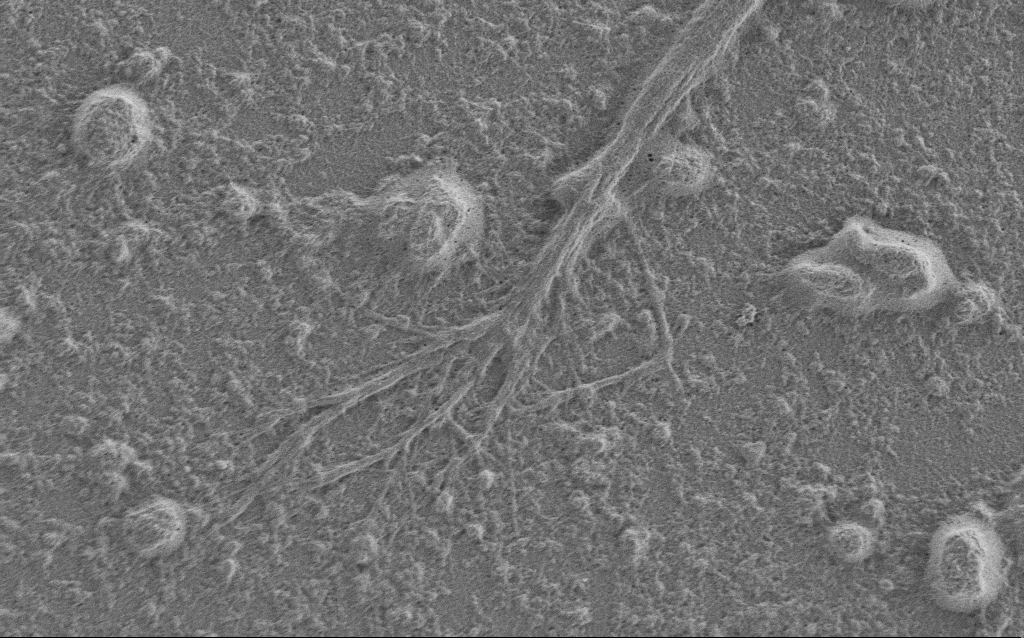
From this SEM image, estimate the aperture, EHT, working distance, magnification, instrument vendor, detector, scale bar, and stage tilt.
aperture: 30 µm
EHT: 1 kV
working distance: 6 mm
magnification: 7.5 K X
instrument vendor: Zeiss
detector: SE2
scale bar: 2000 nm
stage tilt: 0°